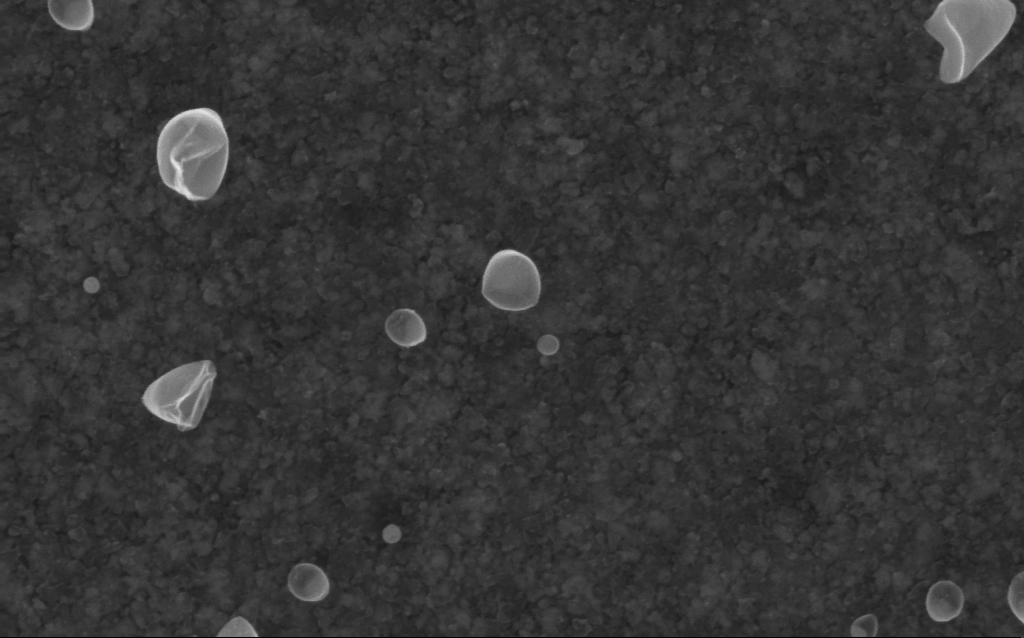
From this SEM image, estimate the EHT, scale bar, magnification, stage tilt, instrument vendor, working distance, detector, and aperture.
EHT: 5 kV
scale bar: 100 nm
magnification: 200 K X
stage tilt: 0°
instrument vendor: Zeiss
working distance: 2.6 mm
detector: InLens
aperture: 30 µm